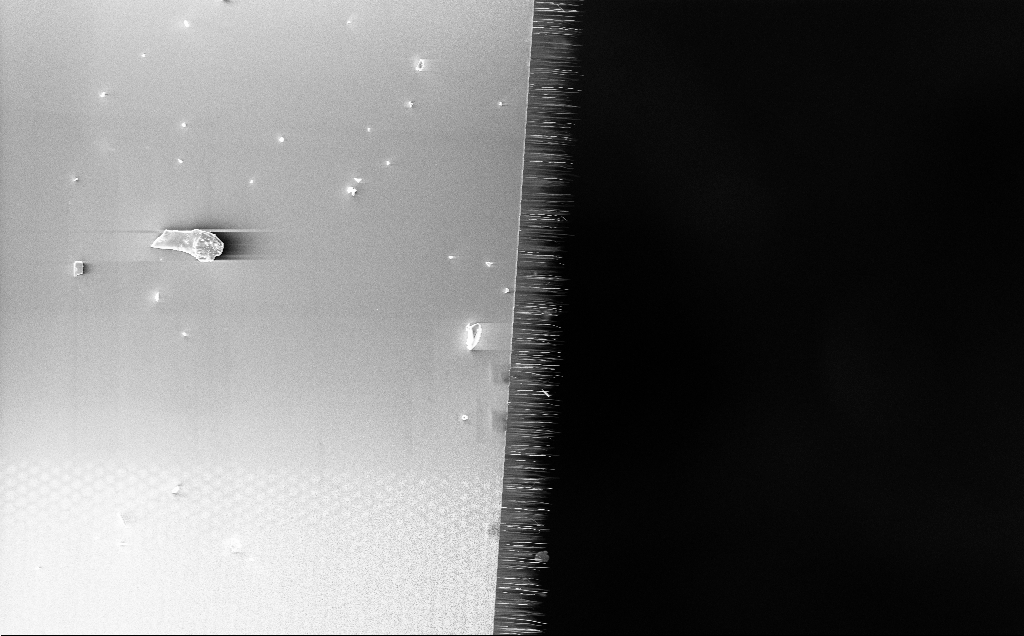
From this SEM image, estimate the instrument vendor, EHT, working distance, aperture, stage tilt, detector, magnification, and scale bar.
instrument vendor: Zeiss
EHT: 5 kV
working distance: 12 mm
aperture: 30 µm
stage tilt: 0°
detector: InLens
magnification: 1.52 K X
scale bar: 10000 nm